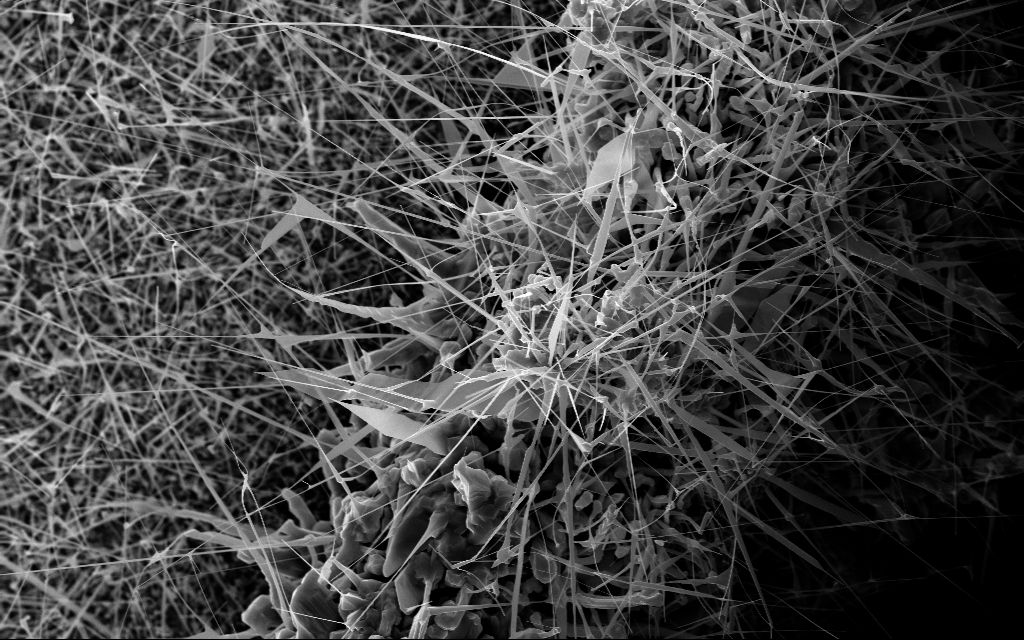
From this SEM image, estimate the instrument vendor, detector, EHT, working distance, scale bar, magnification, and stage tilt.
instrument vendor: Zeiss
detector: InLens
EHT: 10 kV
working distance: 7 mm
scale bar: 2000 nm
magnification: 13.29 K X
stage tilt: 0°